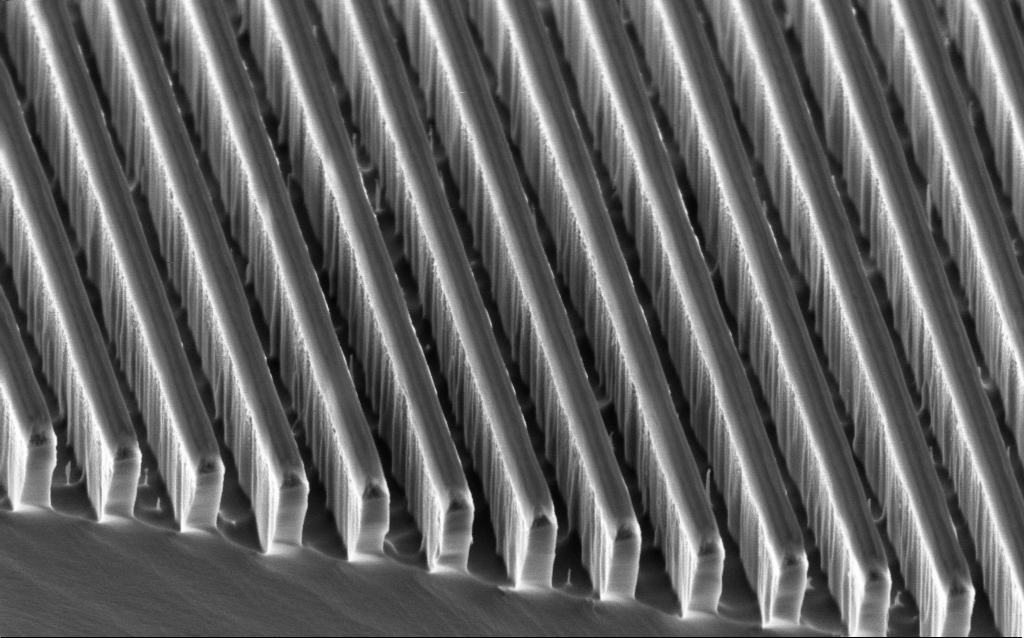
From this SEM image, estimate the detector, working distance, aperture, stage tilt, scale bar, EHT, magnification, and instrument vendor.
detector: InLens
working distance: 3.6 mm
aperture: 30 µm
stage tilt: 45°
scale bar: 1000 nm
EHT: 2 kV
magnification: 54.8 K X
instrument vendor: Zeiss